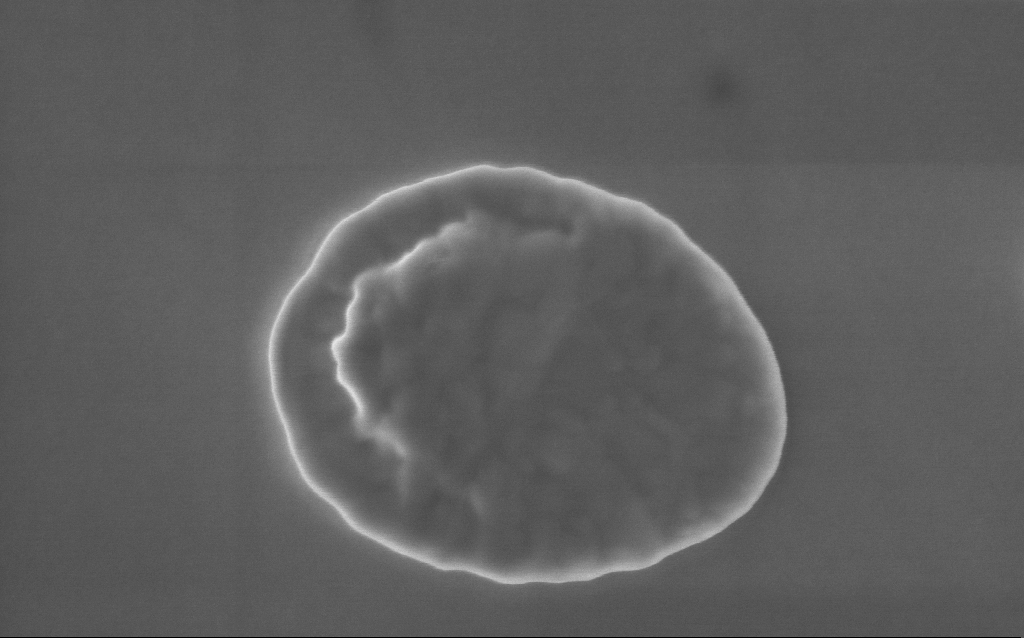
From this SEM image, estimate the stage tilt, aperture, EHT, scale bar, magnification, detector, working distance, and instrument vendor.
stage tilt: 0°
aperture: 30 µm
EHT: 5 kV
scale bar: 200 nm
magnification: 93 K X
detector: InLens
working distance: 3 mm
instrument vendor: Zeiss